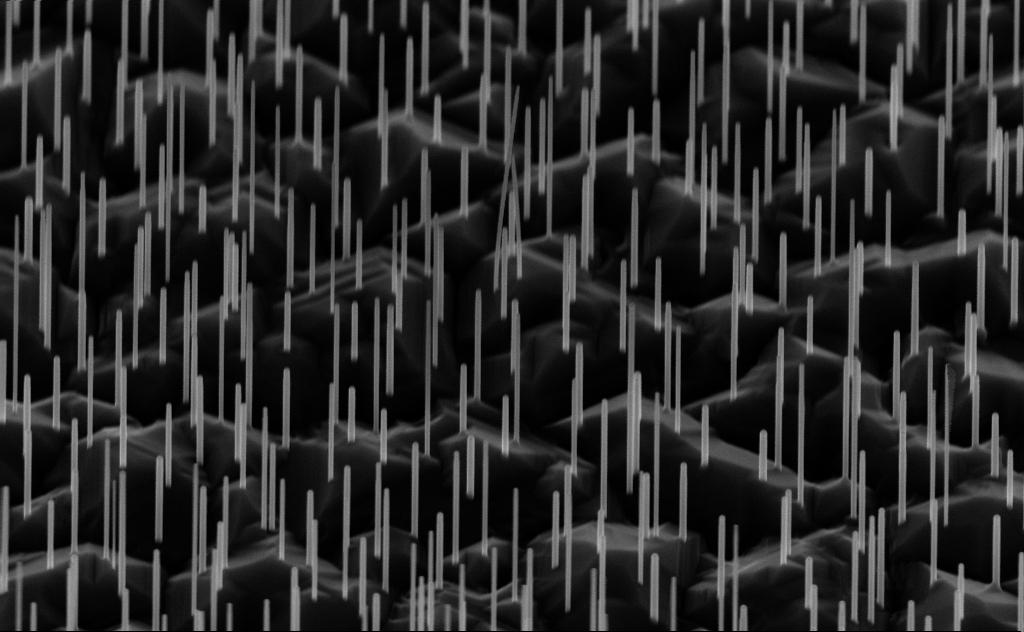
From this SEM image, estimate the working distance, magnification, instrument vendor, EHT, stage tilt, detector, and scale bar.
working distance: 6 mm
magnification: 40 K X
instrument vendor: Zeiss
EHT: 10 kV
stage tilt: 44.2°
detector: InLens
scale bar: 1000 nm